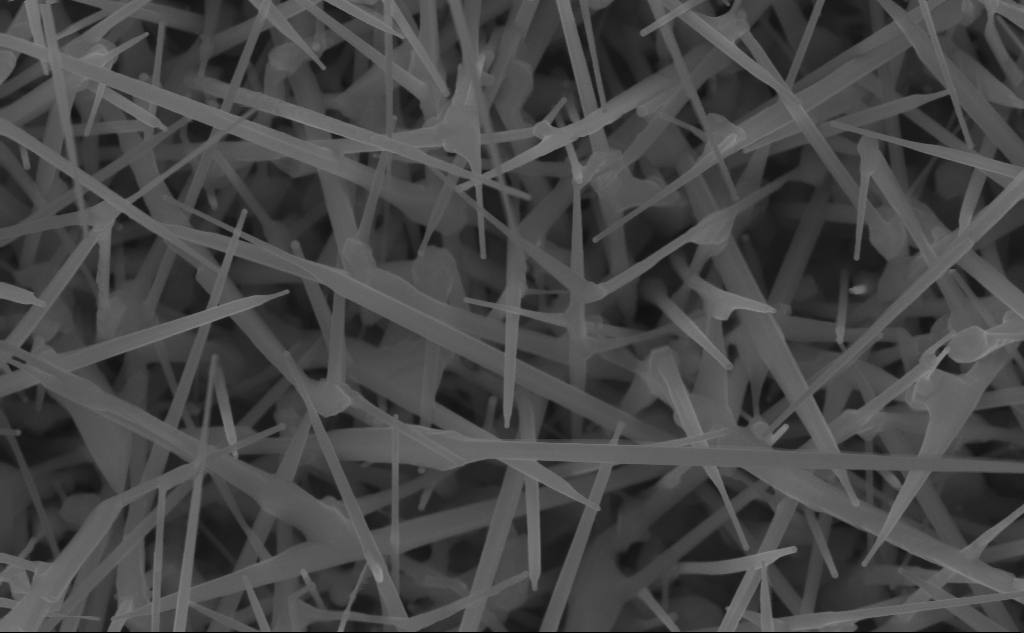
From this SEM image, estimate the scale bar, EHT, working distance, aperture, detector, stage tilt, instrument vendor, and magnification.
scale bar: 200 nm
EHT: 10 kV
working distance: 7 mm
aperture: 30 µm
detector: InLens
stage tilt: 0°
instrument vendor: Zeiss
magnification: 80 K X